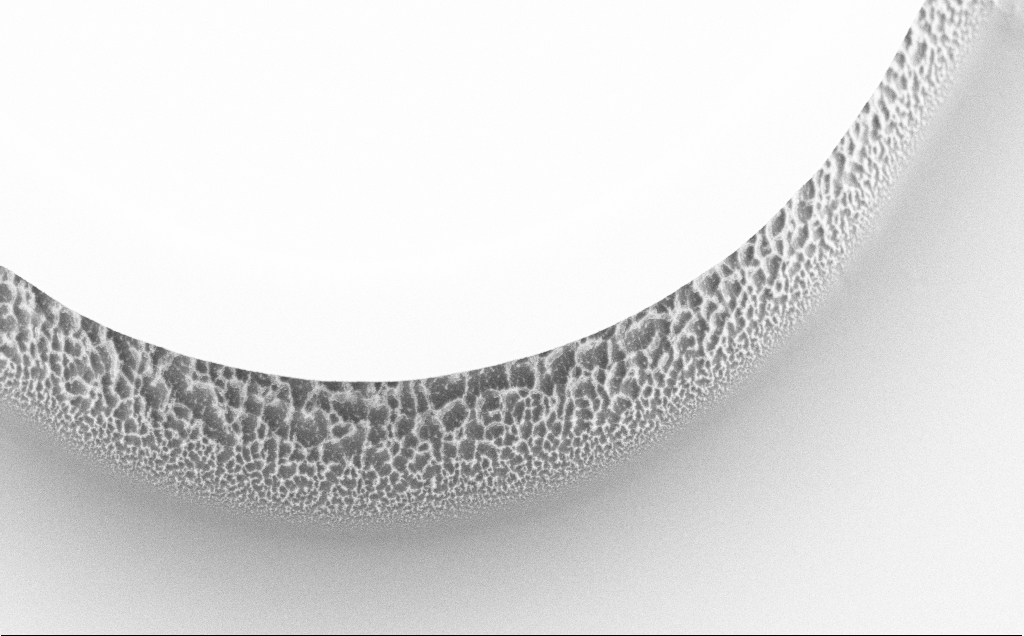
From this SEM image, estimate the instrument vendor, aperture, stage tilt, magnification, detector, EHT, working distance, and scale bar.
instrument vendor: Zeiss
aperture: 30 µm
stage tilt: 45°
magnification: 5.5 K X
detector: SE2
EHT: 5 kV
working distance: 8 mm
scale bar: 10000 nm